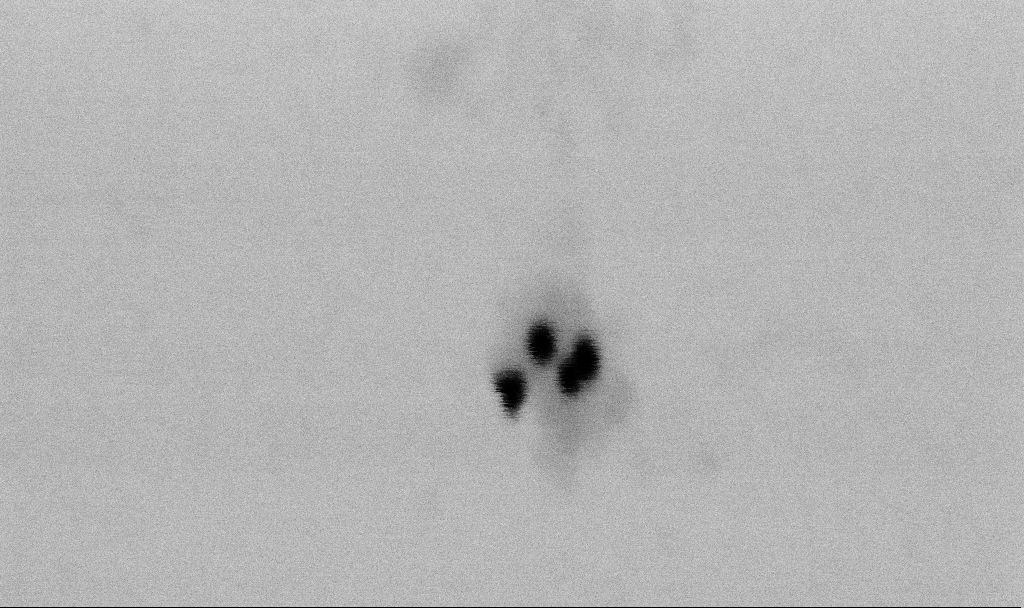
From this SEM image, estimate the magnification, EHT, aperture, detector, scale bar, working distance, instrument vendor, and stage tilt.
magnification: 400 K X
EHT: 2 kV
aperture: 30 µm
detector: SE2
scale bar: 100 nm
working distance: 6.5 mm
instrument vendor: Zeiss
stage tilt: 0°